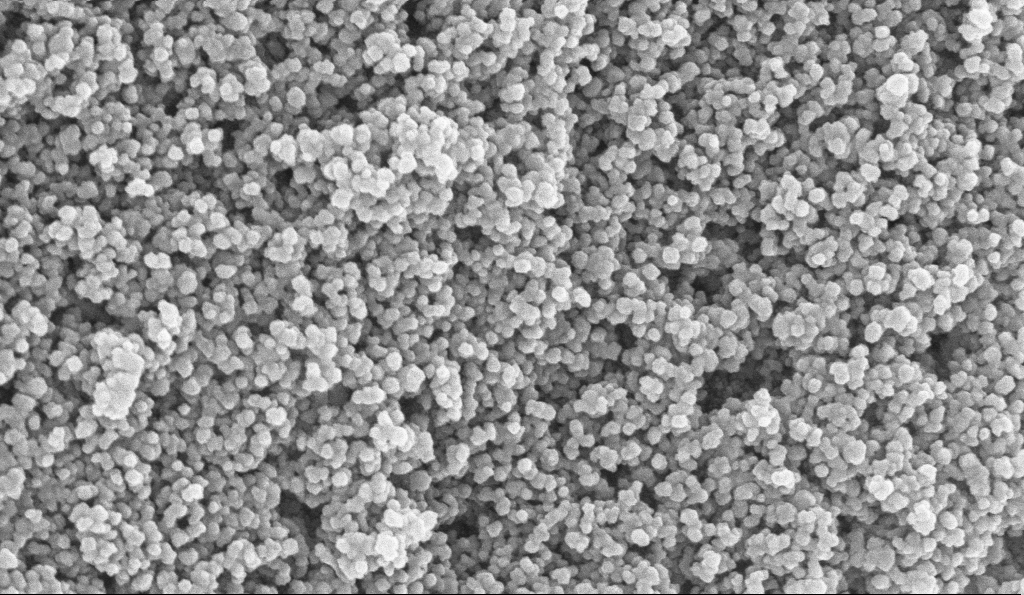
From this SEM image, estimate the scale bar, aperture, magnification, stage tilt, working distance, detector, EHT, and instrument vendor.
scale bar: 100 nm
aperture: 30 µm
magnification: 135 K X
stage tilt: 0°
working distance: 6 mm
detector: InLens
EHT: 10 kV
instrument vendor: Zeiss